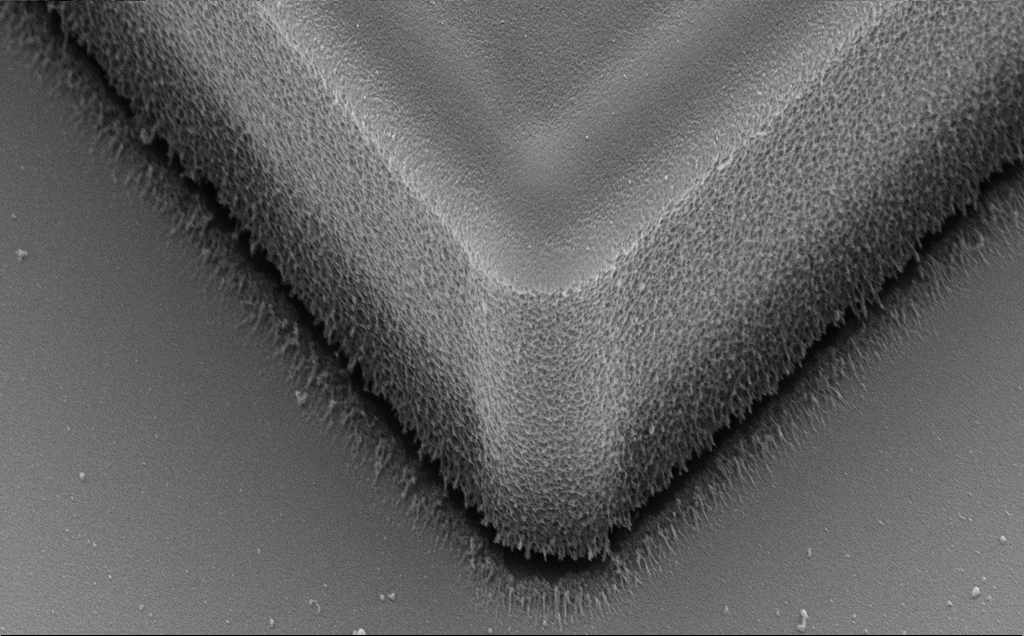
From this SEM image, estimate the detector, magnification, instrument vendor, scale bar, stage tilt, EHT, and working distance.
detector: SE2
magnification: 12.68 K X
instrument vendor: Zeiss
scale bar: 2000 nm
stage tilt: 35.3°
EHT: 10 kV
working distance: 11 mm